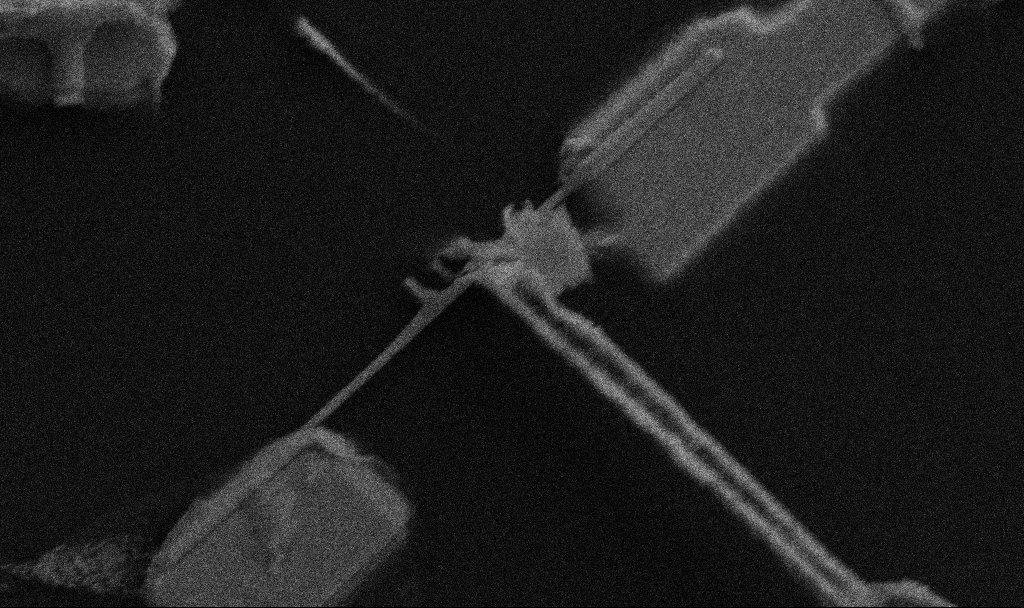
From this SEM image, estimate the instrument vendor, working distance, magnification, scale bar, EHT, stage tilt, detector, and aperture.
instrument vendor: Zeiss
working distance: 10.7 mm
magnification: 42.87 K X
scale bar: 1000 nm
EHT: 5 kV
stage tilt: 0°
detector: SE2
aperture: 30 µm